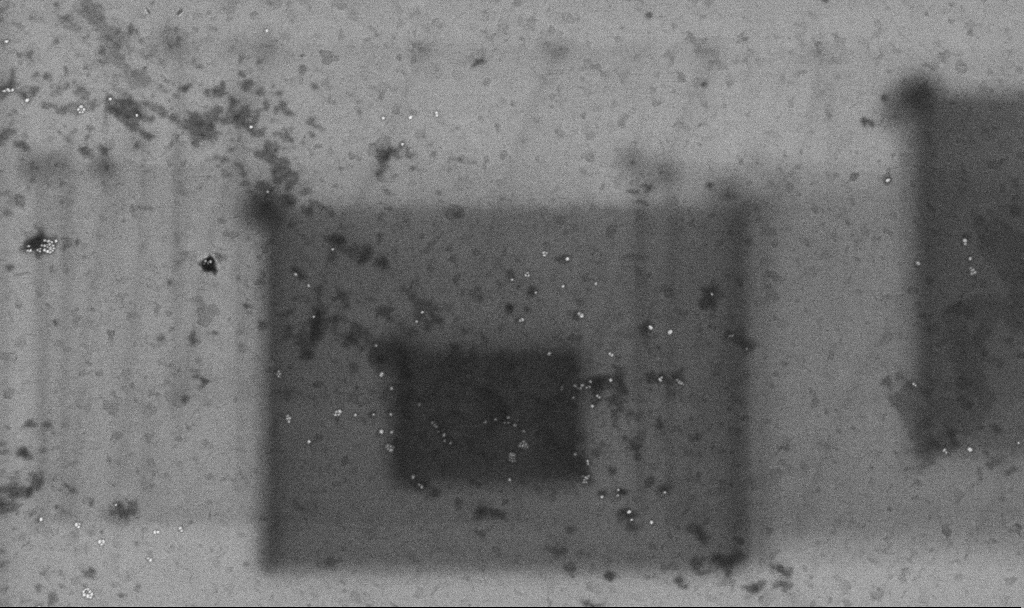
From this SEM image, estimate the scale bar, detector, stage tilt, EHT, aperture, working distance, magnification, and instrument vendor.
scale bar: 1000 nm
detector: InLens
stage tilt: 0°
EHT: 10 kV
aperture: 30 µm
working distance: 3.3 mm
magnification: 50.38 K X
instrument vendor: Zeiss